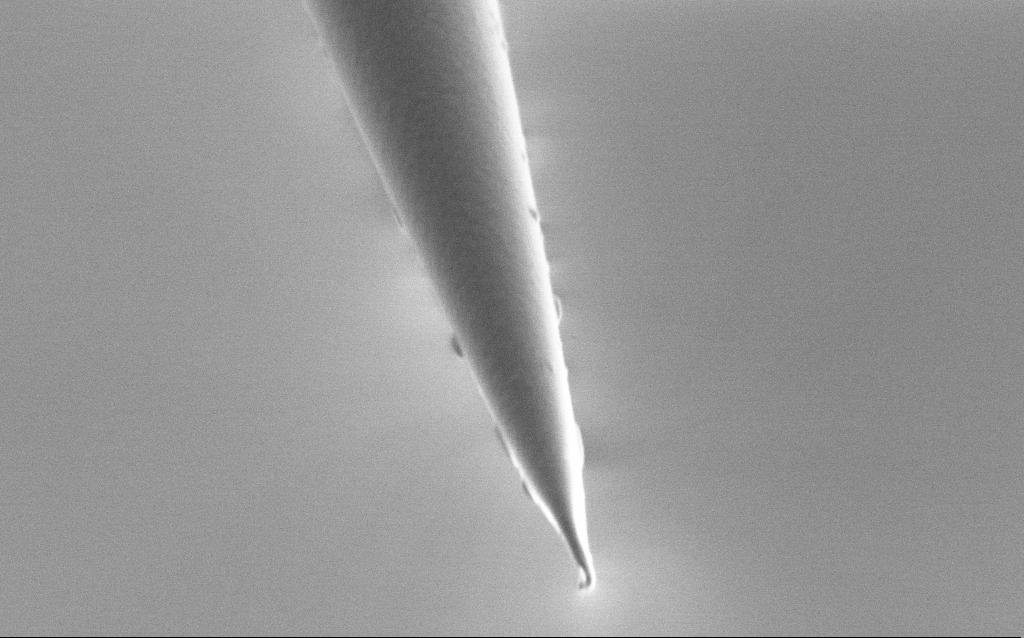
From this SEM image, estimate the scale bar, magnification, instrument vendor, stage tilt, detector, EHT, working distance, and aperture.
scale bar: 1000 nm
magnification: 50 K X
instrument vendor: Zeiss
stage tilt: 45°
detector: SE2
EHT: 1 kV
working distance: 6 mm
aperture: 30 µm